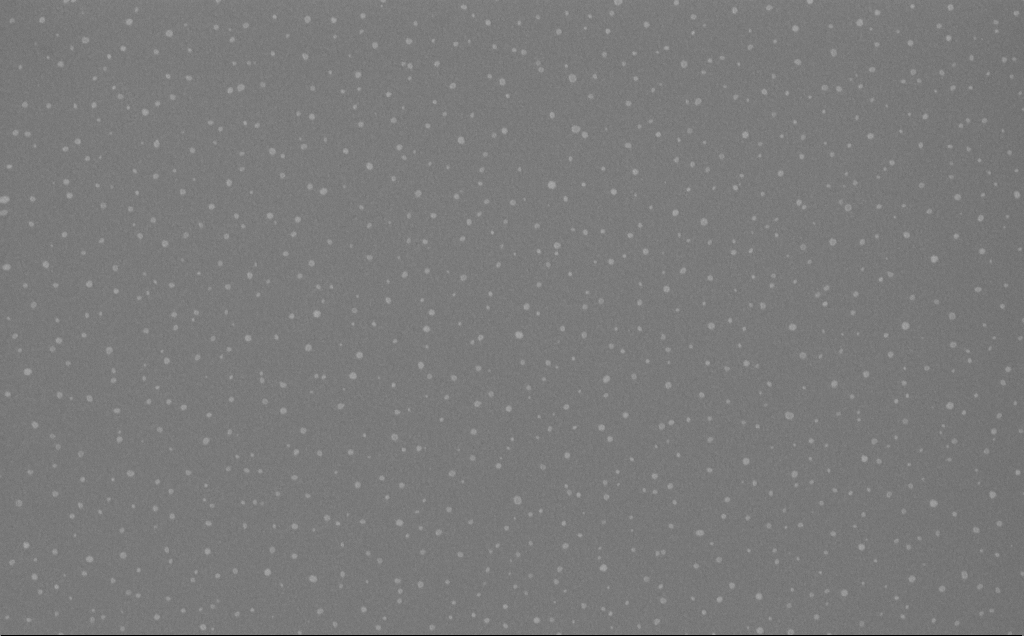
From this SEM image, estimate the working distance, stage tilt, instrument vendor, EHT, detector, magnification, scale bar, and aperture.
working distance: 5 mm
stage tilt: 0°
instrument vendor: Zeiss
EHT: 10 kV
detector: InLens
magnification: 40 K X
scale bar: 1000 nm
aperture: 30 µm